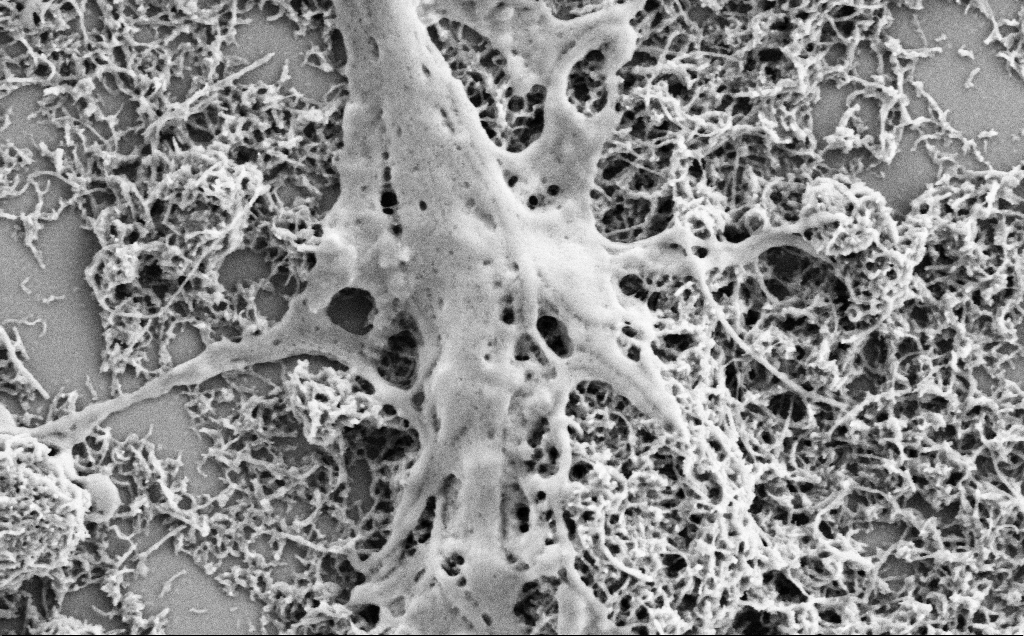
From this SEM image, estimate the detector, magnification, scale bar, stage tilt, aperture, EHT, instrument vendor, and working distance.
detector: SE2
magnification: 40 K X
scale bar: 1000 nm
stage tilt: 0°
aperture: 30 µm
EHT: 2 kV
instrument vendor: Zeiss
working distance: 7.1 mm